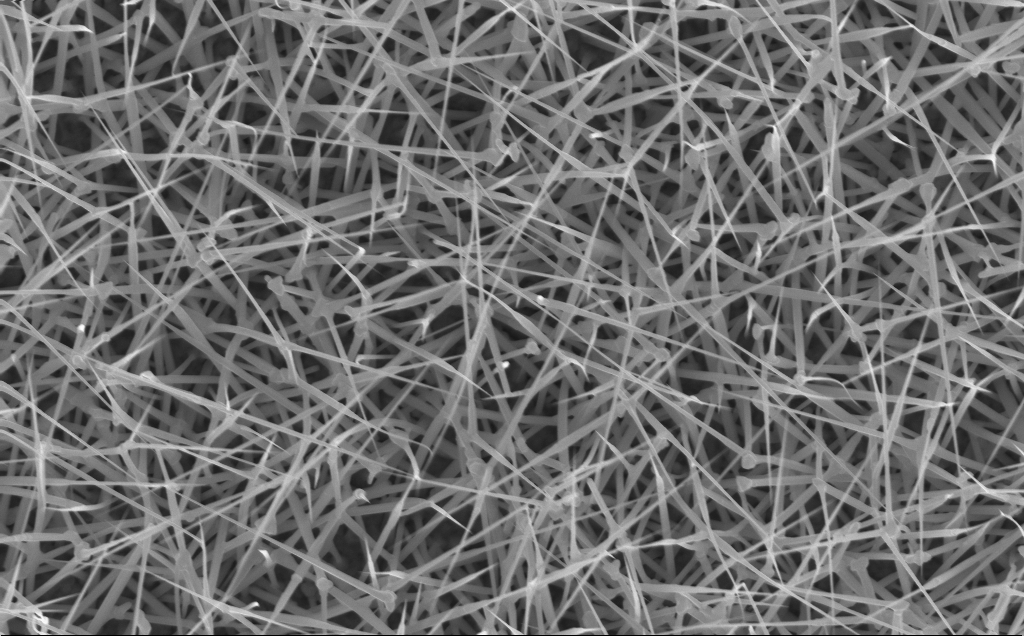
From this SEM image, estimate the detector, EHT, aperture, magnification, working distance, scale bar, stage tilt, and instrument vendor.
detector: InLens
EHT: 10 kV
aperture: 30 µm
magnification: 20 K X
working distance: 6 mm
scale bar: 2000 nm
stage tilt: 0°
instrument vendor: Zeiss